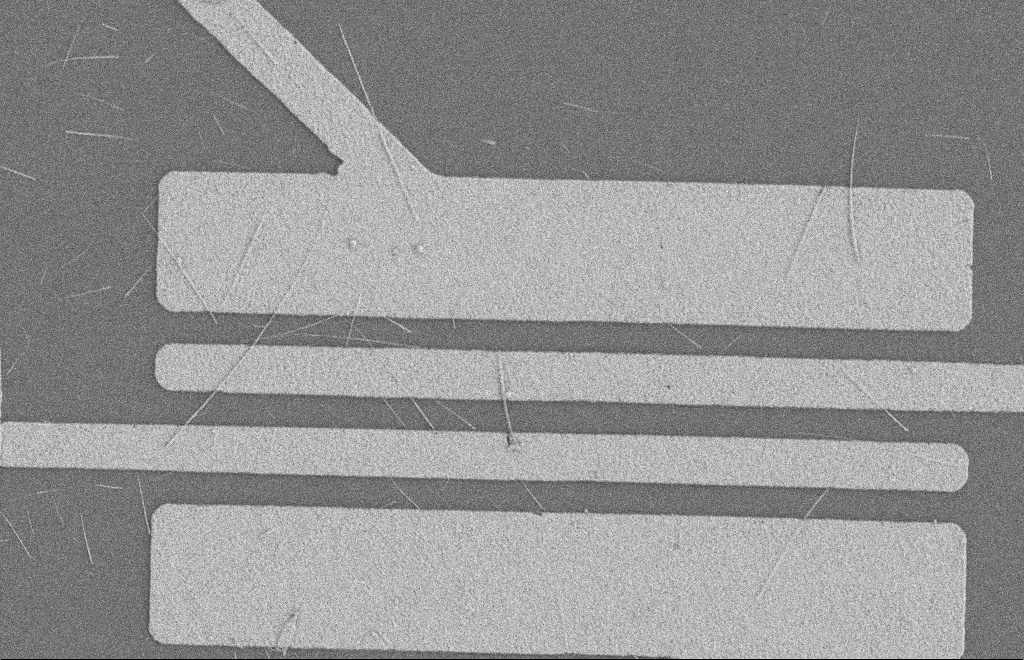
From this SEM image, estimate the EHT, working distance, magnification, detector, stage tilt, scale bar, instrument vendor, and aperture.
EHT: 2 kV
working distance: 8 mm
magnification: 4.9 K X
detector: SE2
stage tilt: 0°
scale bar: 2000 nm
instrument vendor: Zeiss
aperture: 20 µm